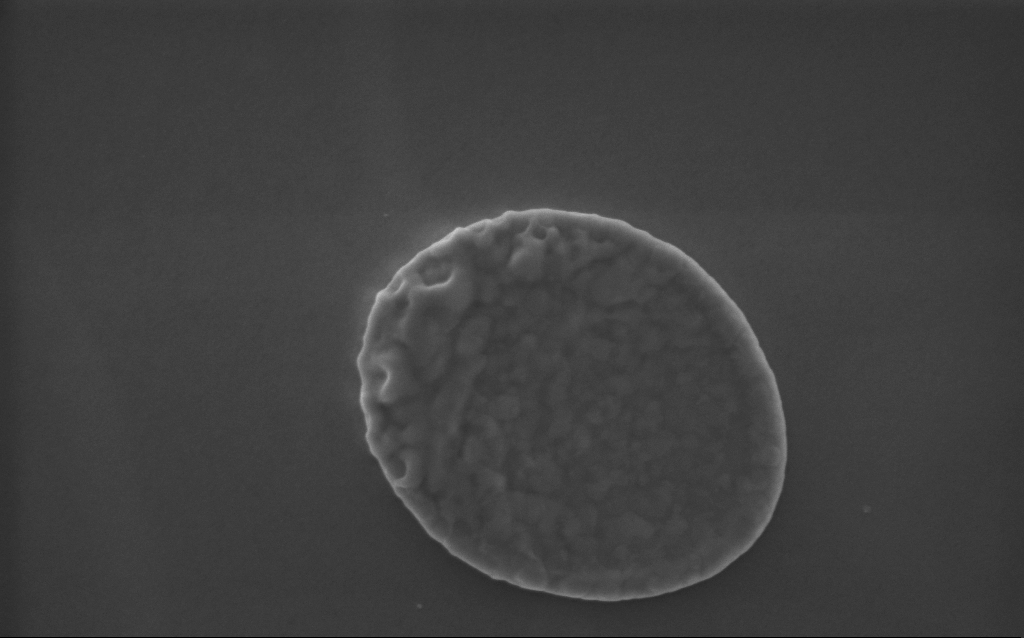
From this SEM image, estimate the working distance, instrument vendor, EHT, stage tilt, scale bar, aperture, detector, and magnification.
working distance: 3 mm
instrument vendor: Zeiss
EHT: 5 kV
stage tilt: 0°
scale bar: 200 nm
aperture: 30 µm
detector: InLens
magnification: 91 K X